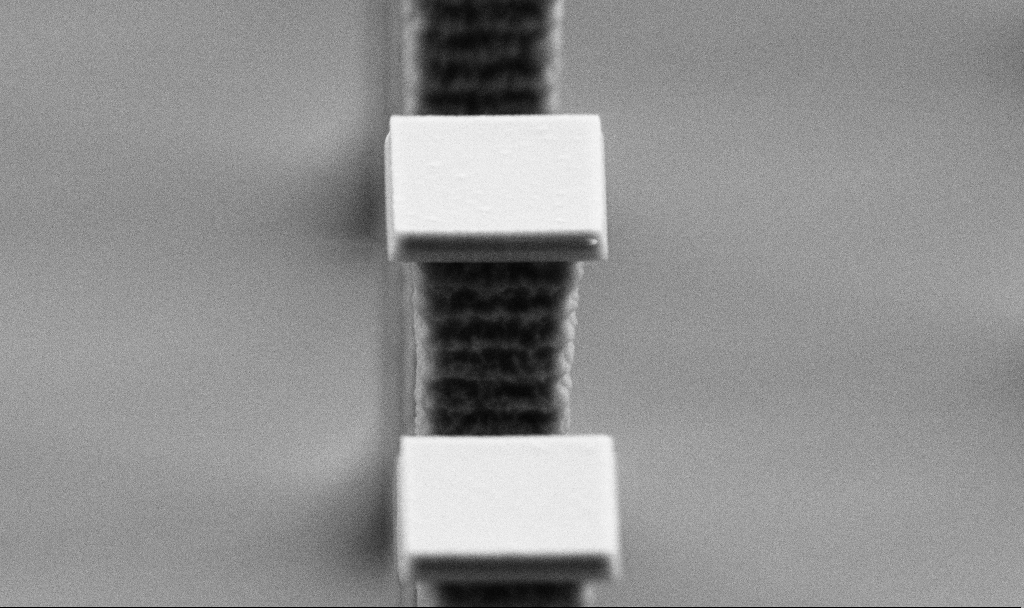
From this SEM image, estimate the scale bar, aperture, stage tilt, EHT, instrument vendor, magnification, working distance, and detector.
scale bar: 1000 nm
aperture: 30 µm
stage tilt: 70°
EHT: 5 kV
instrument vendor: Zeiss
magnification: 26.61 K X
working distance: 5.6 mm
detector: SE2